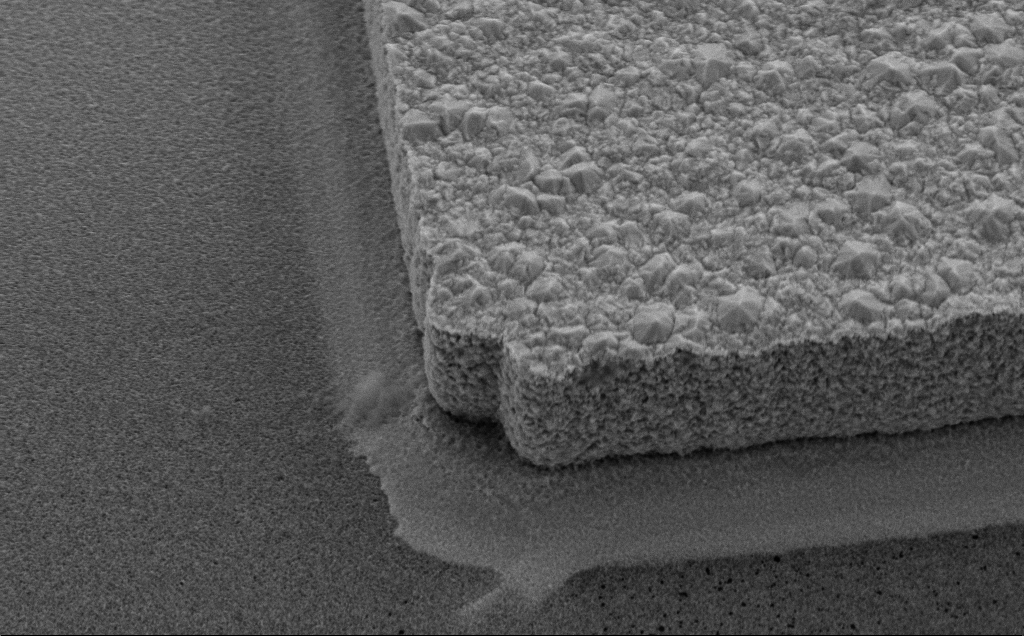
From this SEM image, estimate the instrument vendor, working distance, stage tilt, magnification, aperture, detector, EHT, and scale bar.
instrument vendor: Zeiss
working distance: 8 mm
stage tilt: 35°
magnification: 16.21 K X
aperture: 30 µm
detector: SE2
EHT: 10 kV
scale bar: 2000 nm